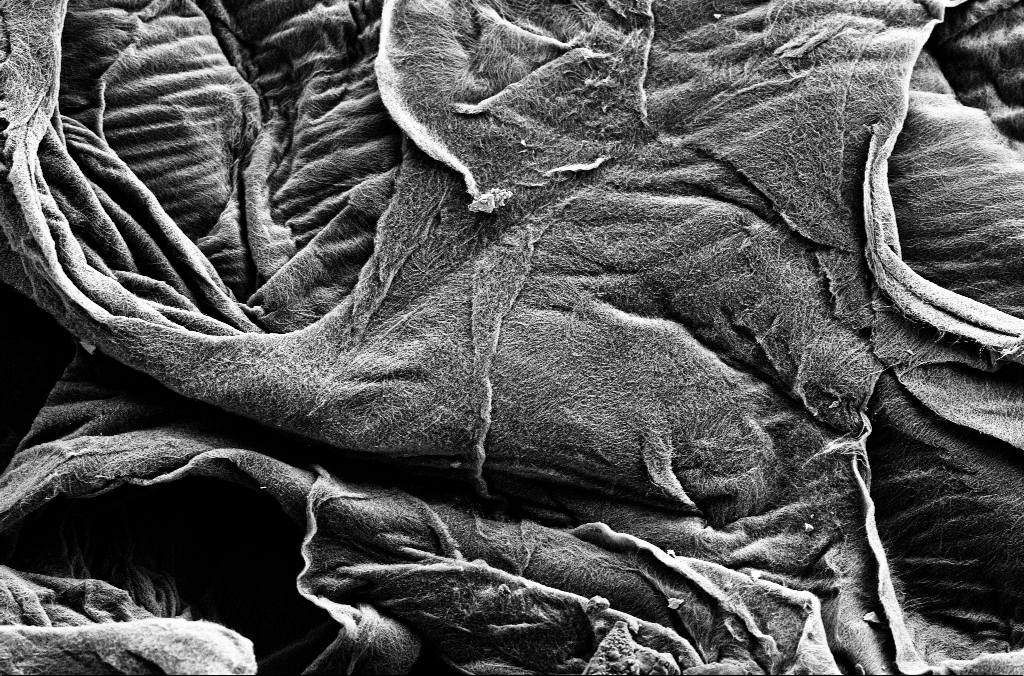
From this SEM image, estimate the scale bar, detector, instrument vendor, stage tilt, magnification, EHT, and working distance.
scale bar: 10000 nm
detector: SE2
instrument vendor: Zeiss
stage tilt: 0°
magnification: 5 K X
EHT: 1.8 kV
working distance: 5.4 mm